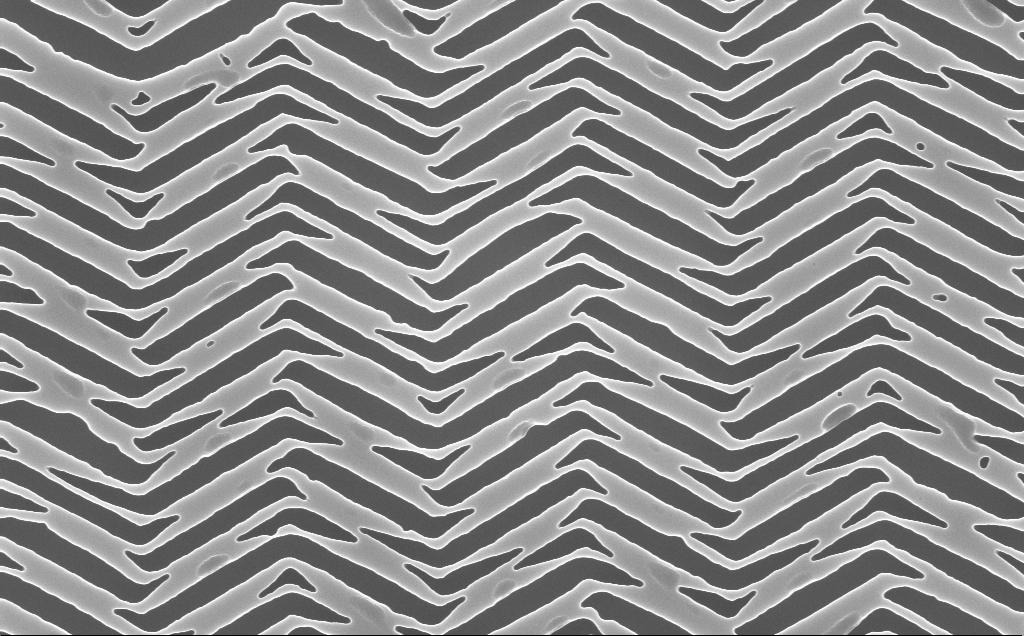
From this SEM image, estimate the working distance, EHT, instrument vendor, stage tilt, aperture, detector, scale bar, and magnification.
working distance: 4 mm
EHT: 10 kV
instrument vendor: Zeiss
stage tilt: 0°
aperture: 30 µm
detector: InLens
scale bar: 1000 nm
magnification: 54.35 K X